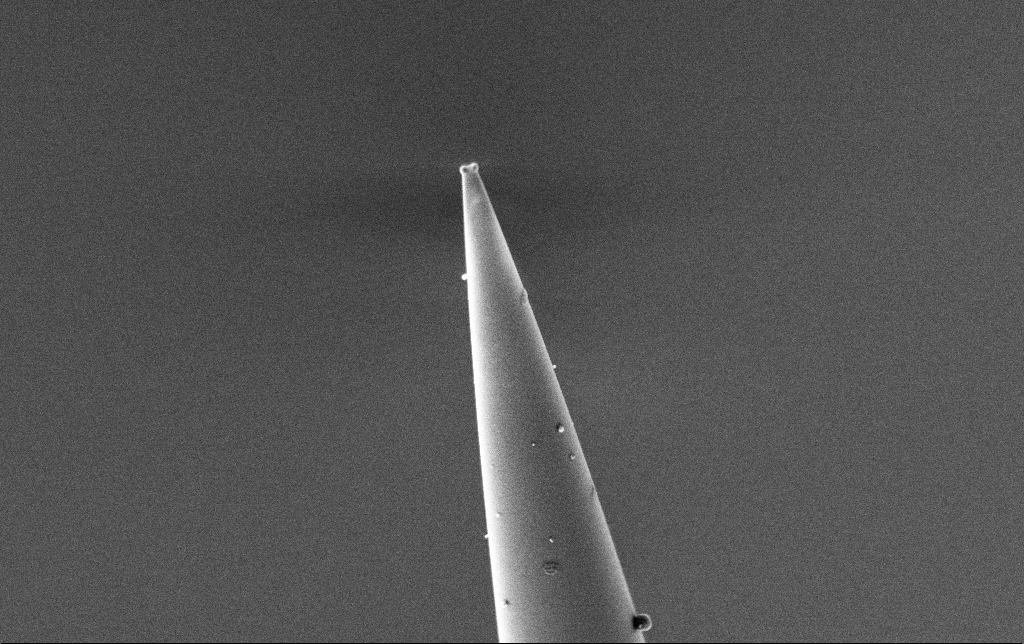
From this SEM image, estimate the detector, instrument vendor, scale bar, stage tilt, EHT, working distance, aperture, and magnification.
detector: SE2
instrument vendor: Zeiss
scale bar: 2000 nm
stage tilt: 45°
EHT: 2 kV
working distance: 7.6 mm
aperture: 30 µm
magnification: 20 K X